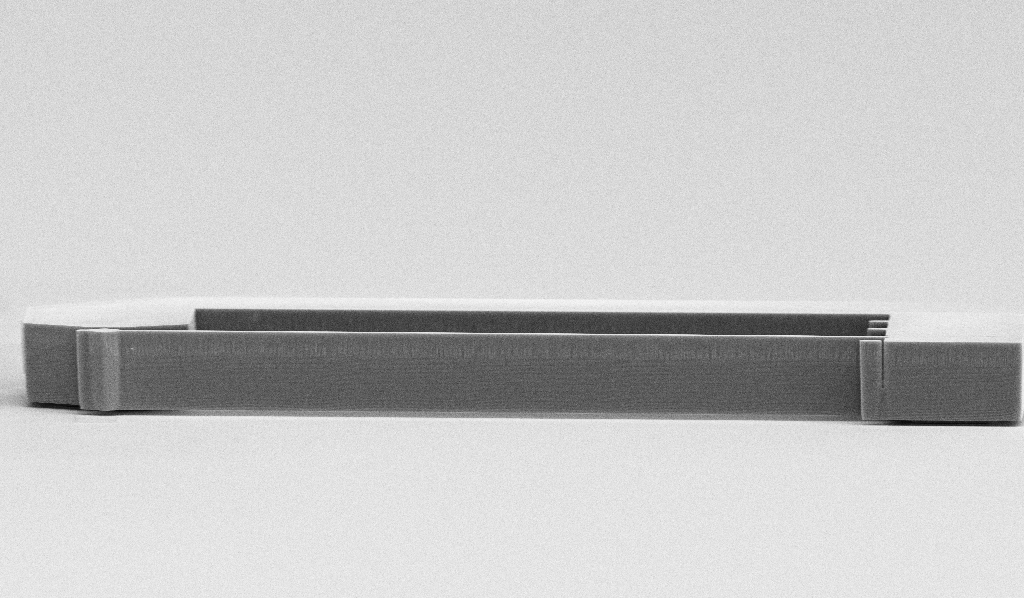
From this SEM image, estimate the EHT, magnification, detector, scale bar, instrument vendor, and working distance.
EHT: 10 kV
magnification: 2.01 K X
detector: InLens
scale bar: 10000 nm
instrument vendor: Zeiss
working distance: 14.3 mm